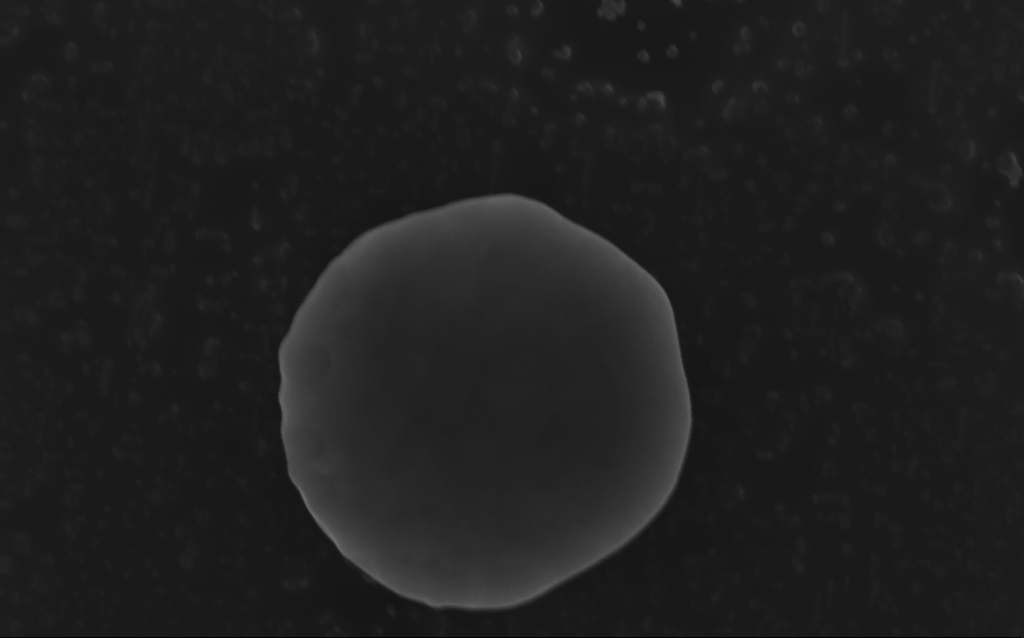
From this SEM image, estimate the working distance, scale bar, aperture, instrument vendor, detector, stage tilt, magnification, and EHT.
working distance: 5 mm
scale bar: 200 nm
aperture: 30 µm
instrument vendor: Zeiss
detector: InLens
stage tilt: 0°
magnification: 164.38 K X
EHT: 10 kV